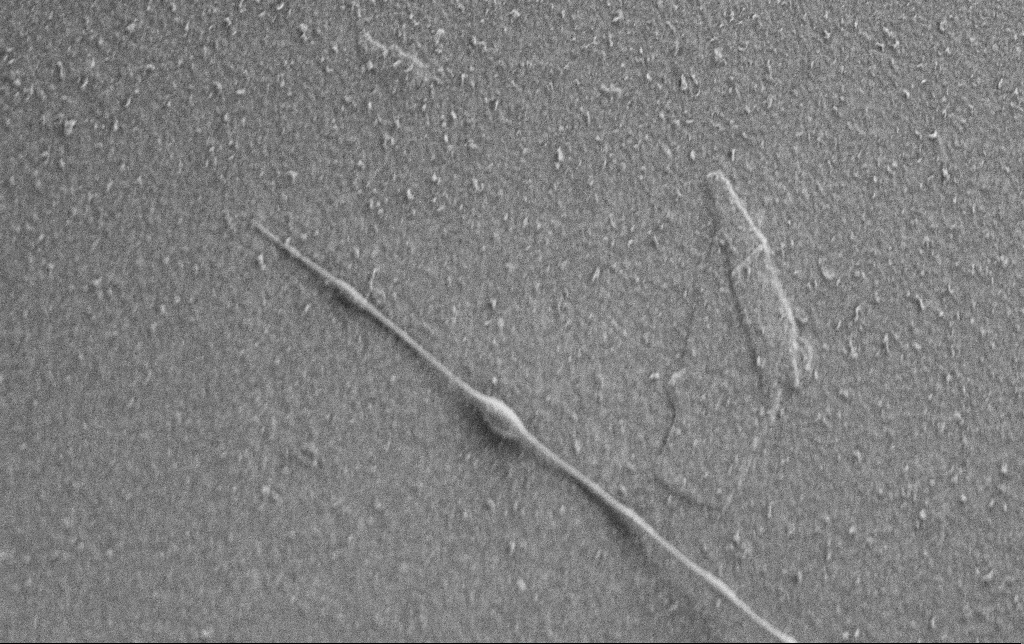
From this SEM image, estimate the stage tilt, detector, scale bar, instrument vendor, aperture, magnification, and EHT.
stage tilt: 0°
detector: SE2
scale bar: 2000 nm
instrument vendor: Zeiss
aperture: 30 µm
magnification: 10 K X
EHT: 0.9 kV